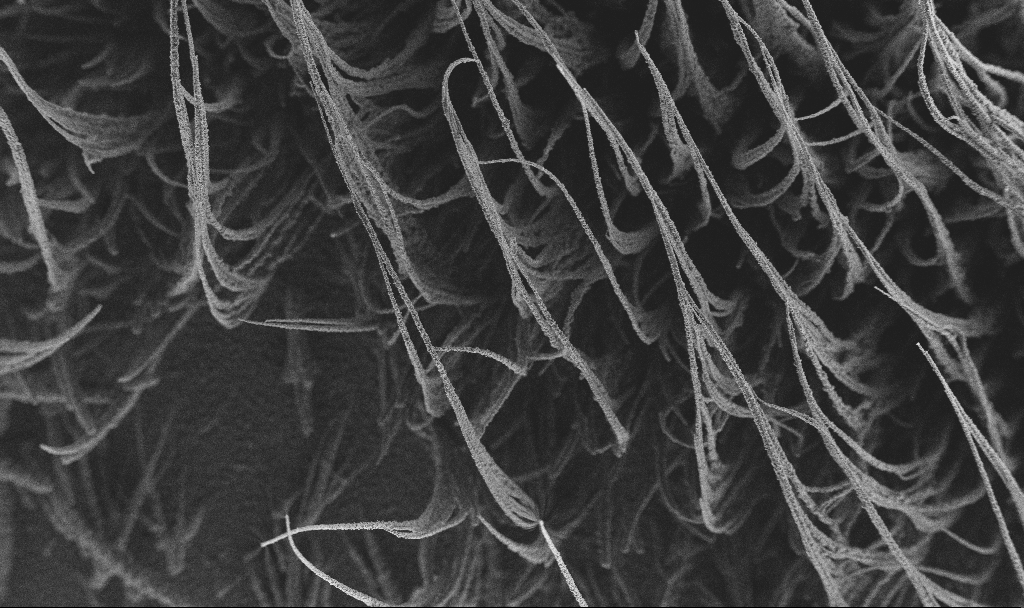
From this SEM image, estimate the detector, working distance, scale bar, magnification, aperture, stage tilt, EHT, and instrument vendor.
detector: SE2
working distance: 2.9 mm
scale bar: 100000 nm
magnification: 0.15 K X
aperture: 30 µm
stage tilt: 0°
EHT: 3 kV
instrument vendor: Zeiss